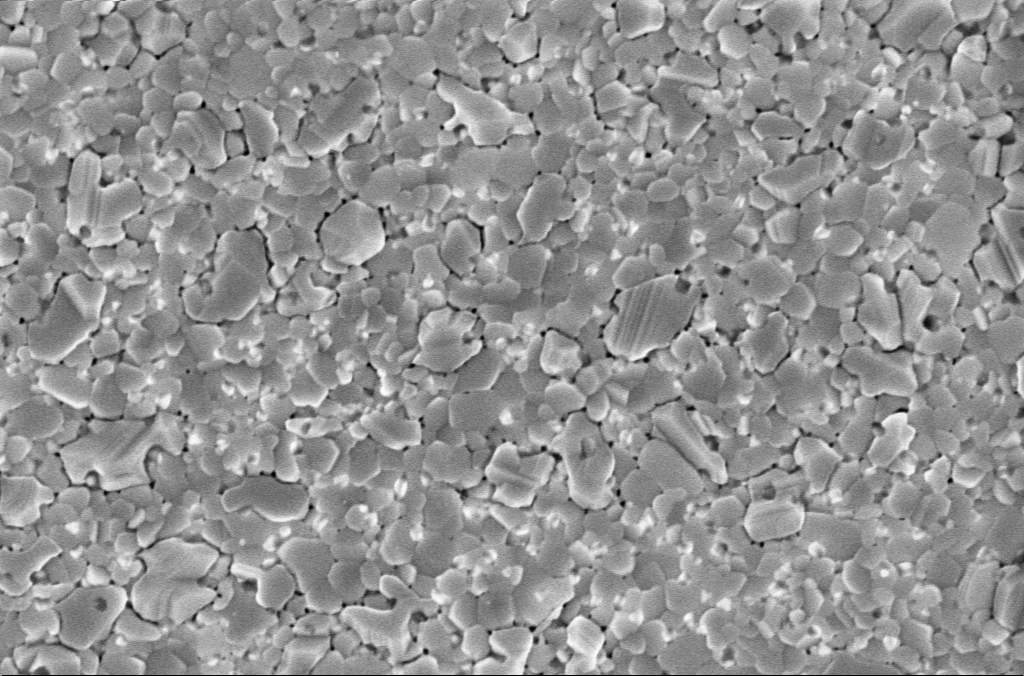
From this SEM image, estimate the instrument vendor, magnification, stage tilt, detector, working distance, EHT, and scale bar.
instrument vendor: Zeiss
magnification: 50 K X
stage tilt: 0°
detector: InLens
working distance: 3 mm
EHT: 5 kV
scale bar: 1000 nm